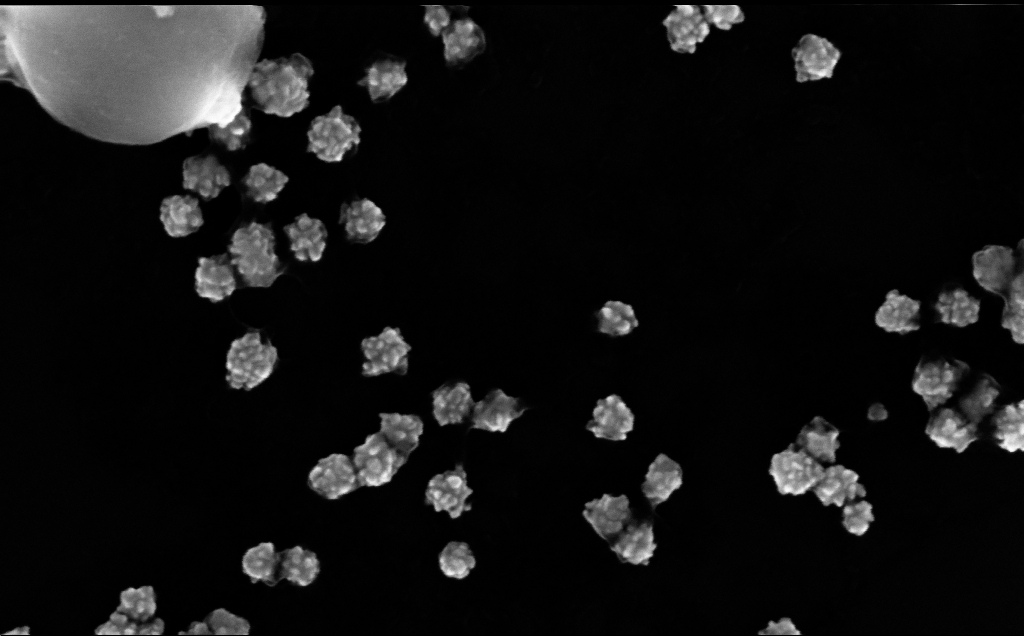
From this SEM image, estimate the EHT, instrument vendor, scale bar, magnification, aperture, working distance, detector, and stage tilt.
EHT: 10 kV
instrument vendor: Zeiss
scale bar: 200 nm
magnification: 247.55 K X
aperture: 30 µm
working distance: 4 mm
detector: InLens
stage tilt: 0°